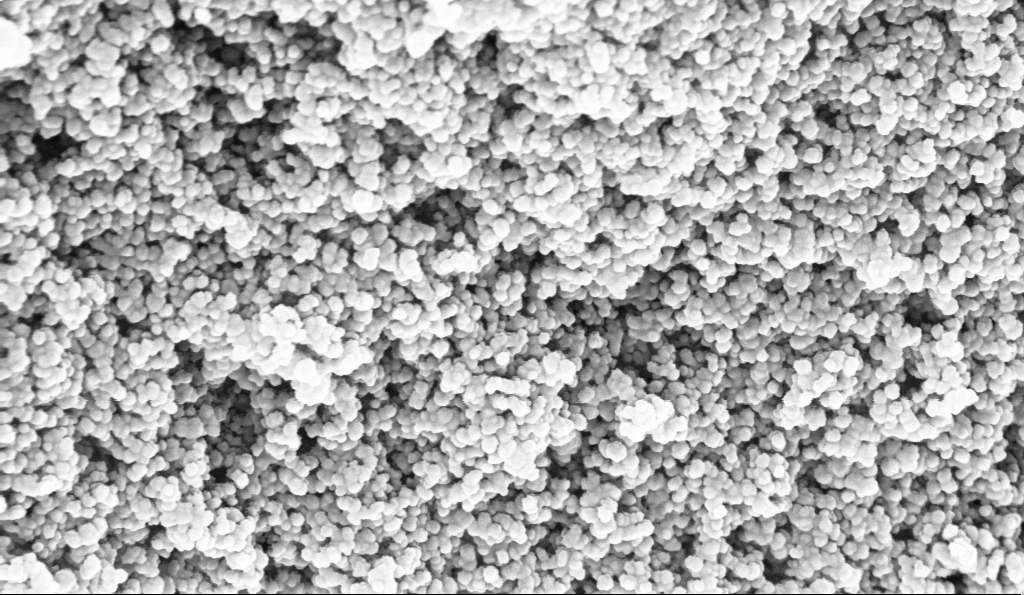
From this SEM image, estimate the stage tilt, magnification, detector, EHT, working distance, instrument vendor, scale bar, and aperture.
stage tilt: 0°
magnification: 135 K X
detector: InLens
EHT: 10 kV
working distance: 5.1 mm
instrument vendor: Zeiss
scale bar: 200 nm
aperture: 30 µm